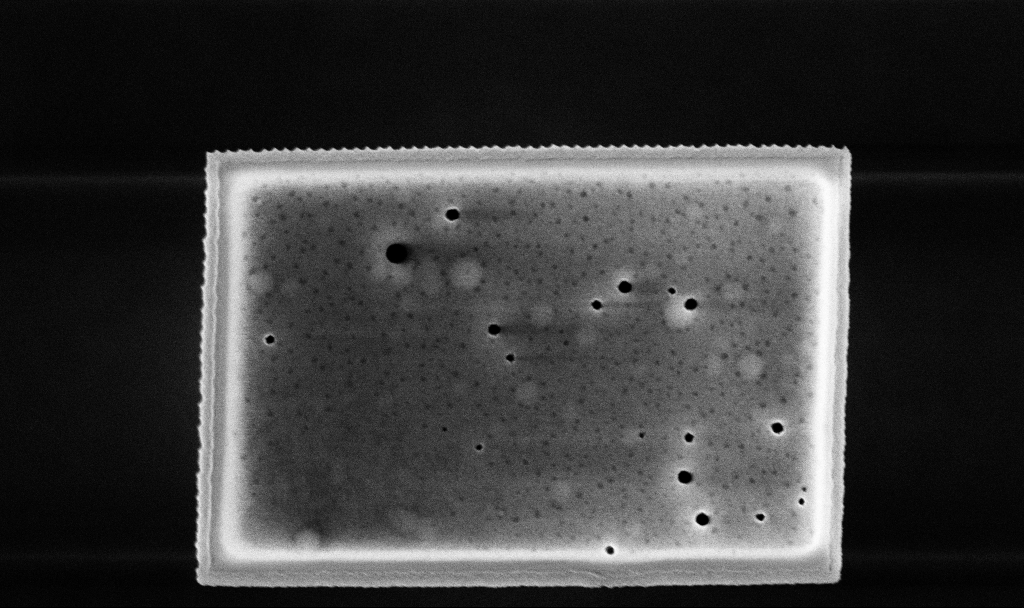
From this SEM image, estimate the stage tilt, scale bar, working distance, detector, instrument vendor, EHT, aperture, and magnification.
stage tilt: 0°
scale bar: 1000 nm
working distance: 3.3 mm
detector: InLens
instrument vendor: Zeiss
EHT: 5 kV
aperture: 30 µm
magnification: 54.73 K X